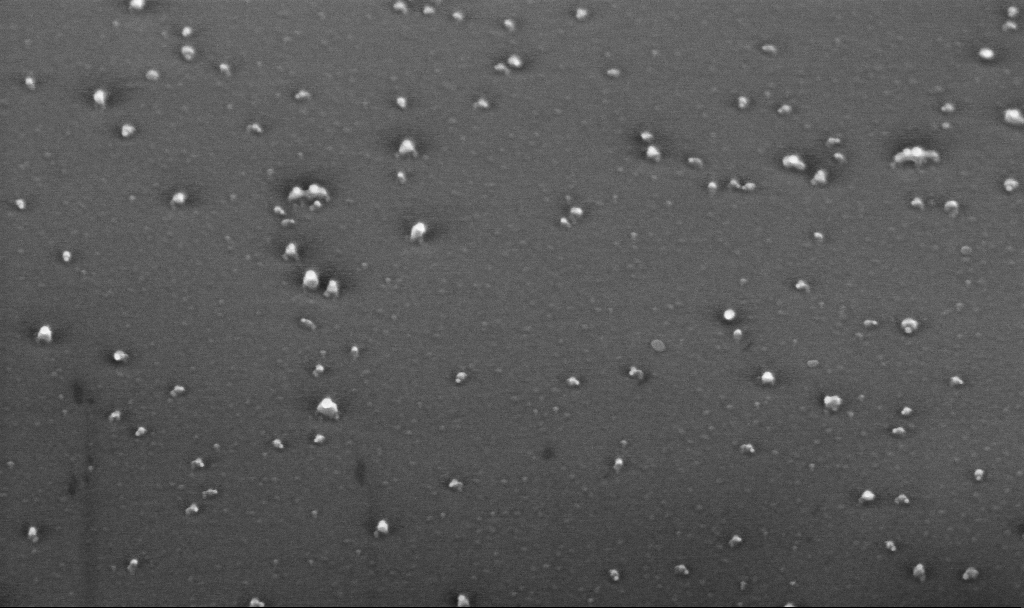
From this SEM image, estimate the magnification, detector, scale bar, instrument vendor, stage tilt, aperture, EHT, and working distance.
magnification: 100 K X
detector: InLens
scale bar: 200 nm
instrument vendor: Zeiss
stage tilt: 45°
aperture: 30 µm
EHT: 10 kV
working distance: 4.4 mm